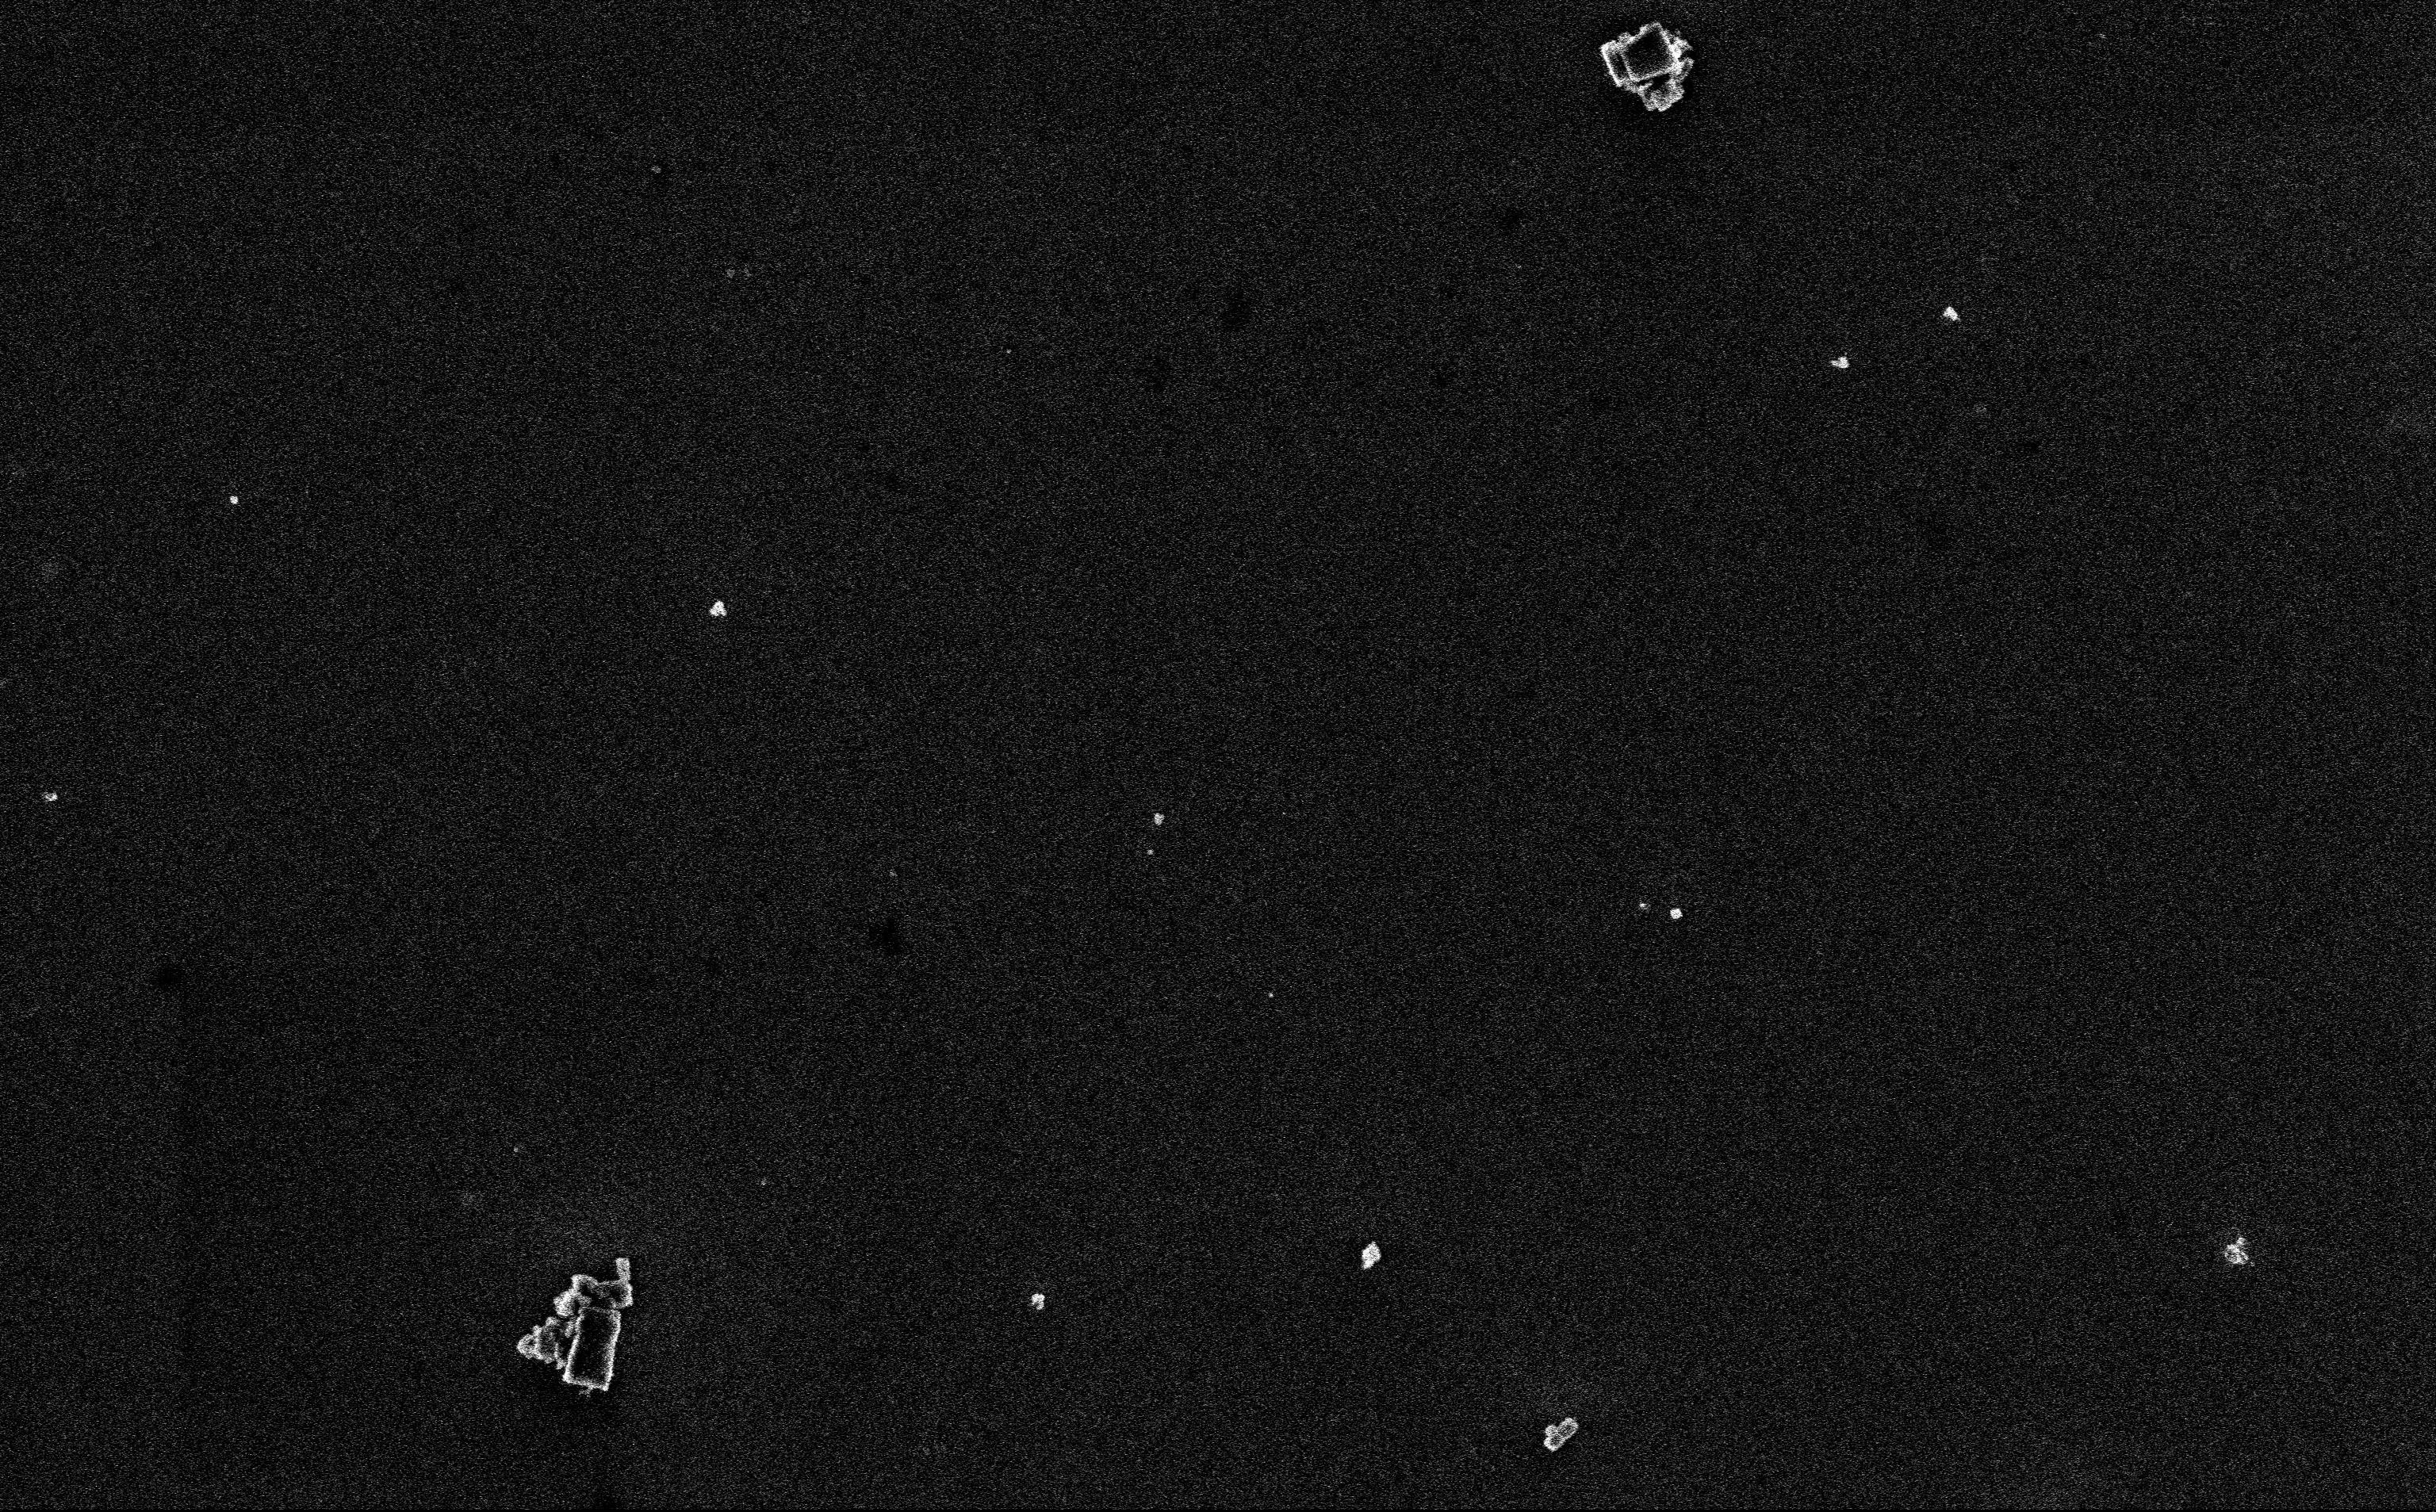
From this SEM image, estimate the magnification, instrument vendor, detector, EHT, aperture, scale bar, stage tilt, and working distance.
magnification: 12.85 K X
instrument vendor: Zeiss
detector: InLens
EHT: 3 kV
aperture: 30 µm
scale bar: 2000 nm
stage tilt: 0°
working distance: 3 mm